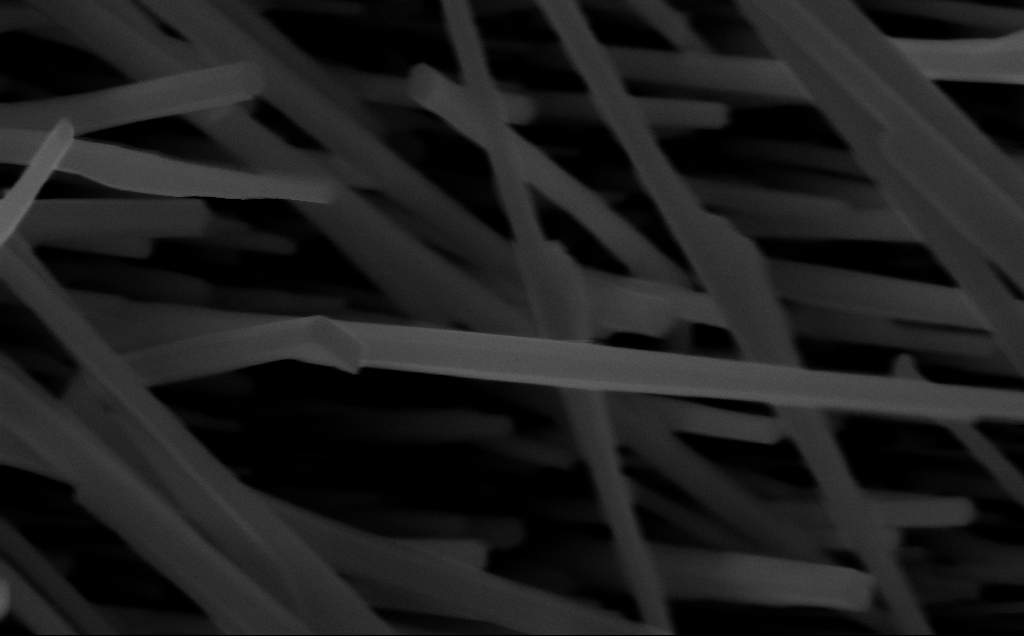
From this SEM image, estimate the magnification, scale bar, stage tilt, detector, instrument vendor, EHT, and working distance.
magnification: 92.32 K X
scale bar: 200 nm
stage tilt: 0°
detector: InLens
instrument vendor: Zeiss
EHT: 10 kV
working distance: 6 mm